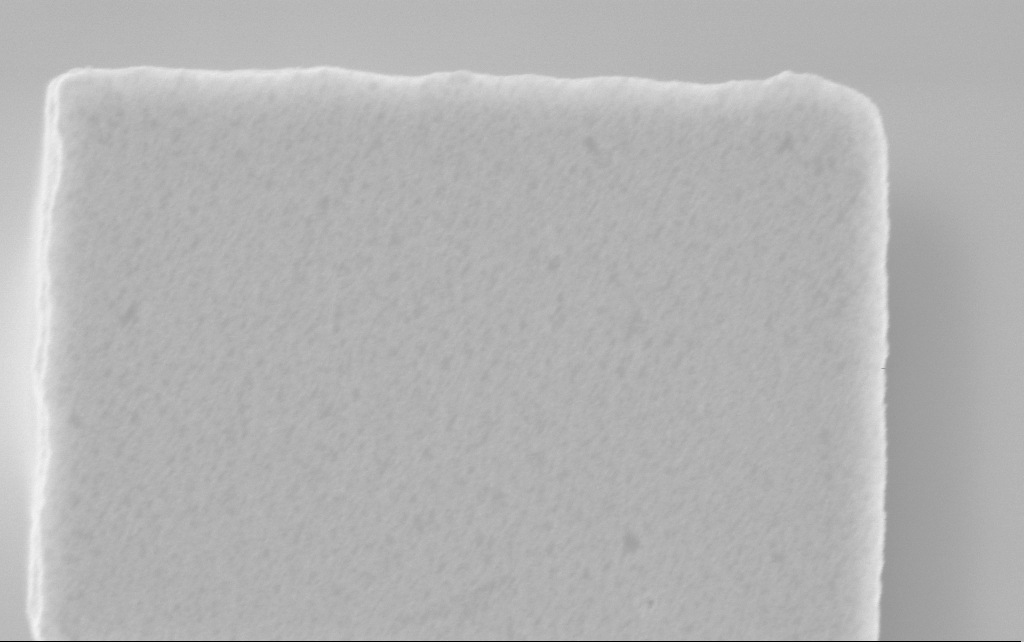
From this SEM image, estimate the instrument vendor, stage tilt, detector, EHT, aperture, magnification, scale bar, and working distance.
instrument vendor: Zeiss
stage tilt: -0°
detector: InLens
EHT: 3 kV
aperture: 30 µm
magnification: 102.83 K X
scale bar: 200 nm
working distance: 2.5 mm